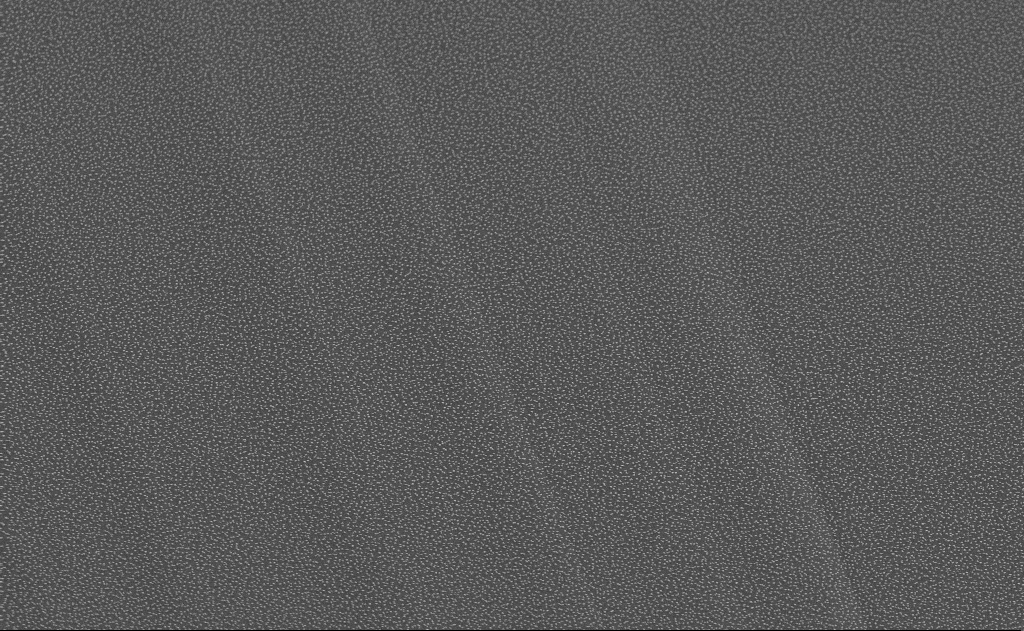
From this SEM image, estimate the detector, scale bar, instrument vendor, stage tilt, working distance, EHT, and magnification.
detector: SE2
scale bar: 2000 nm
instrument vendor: Zeiss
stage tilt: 32°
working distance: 12 mm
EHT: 10 kV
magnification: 10 K X